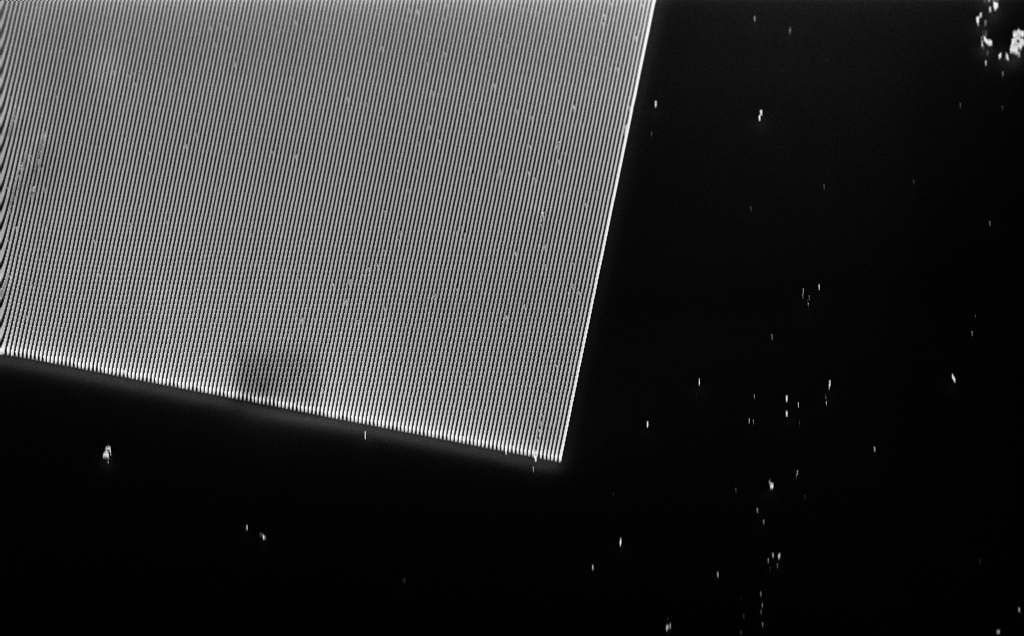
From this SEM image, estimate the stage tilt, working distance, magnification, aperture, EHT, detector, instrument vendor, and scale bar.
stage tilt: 45°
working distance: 6 mm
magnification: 4.84 K X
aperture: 30 µm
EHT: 10 kV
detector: InLens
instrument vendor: Zeiss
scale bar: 10000 nm